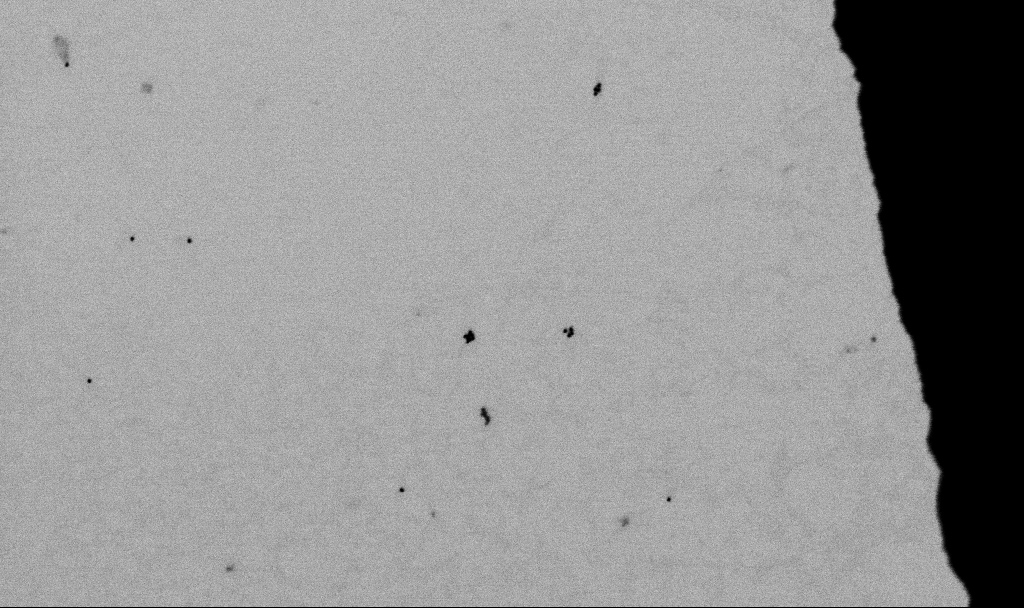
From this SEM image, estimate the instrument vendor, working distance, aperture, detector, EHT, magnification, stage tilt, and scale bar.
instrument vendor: Zeiss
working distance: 5.5 mm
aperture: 30 µm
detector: SE2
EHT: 2 kV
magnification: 60 K X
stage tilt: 0°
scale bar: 1000 nm